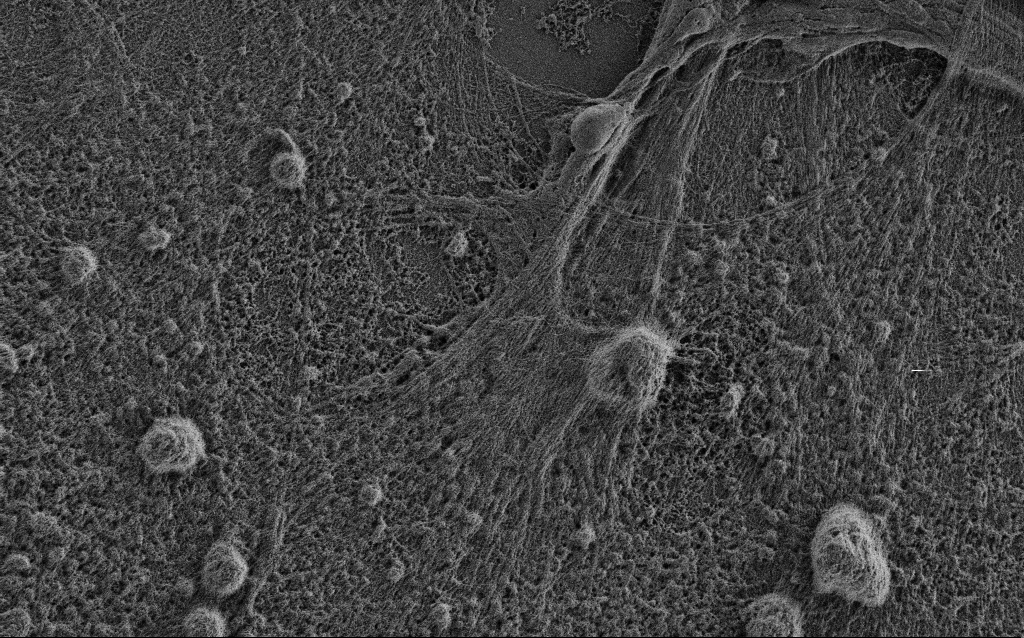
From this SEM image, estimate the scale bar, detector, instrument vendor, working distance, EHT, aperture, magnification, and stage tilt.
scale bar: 2000 nm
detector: SE2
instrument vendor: Zeiss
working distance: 3.4 mm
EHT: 0.9 kV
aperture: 30 µm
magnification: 10 K X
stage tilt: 0°